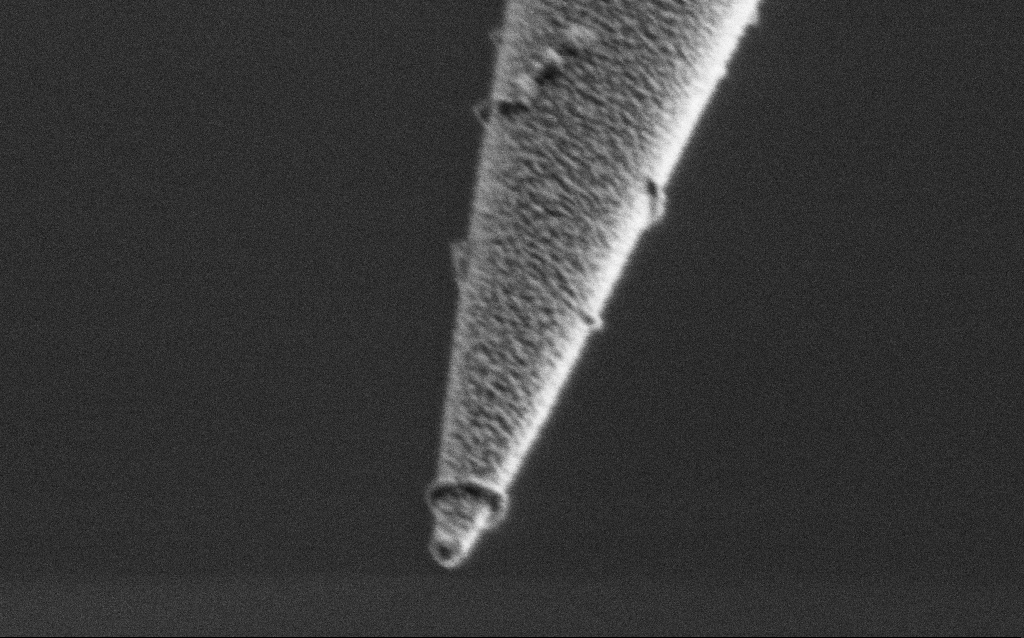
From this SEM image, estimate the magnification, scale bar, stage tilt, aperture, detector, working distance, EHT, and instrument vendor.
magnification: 100 K X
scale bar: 200 nm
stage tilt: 45°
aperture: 30 µm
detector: SE2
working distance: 6.5 mm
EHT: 1 kV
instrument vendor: Zeiss